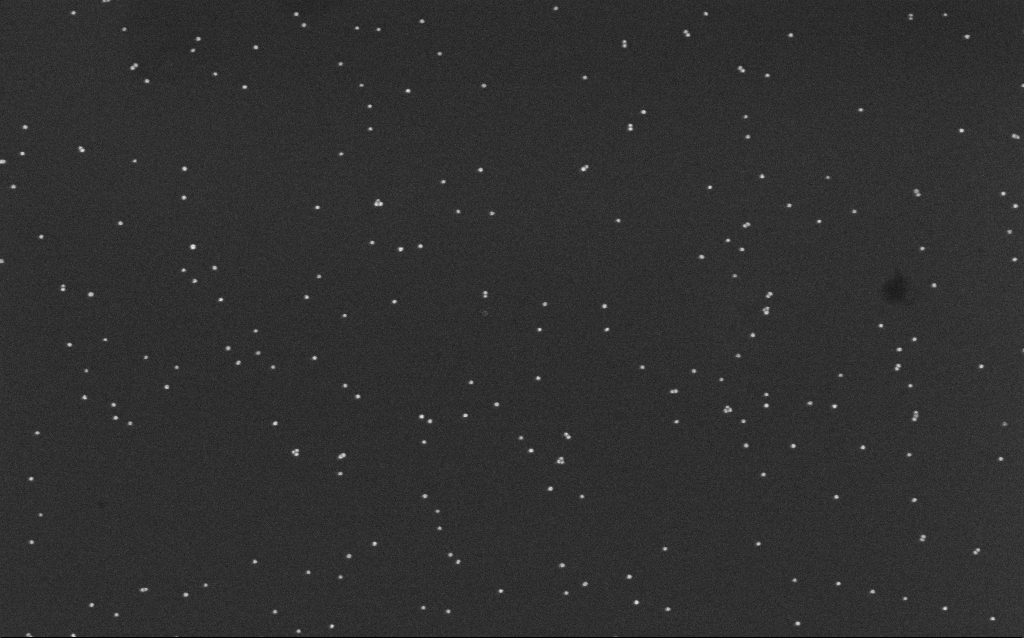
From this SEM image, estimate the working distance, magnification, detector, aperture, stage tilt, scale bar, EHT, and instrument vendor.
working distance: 6.6 mm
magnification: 100 K X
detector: InLens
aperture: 30 µm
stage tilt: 0°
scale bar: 200 nm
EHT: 10 kV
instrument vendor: Zeiss